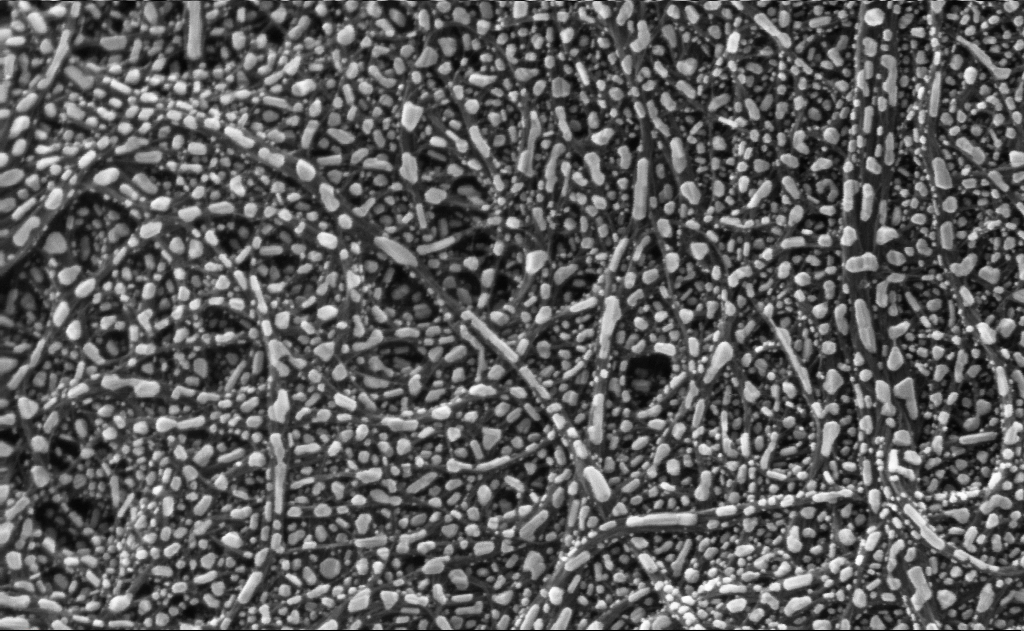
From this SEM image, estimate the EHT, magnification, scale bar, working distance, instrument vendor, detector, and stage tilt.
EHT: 10 kV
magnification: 247.11 K X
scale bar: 100 nm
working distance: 3 mm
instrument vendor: Zeiss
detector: InLens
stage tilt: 0°